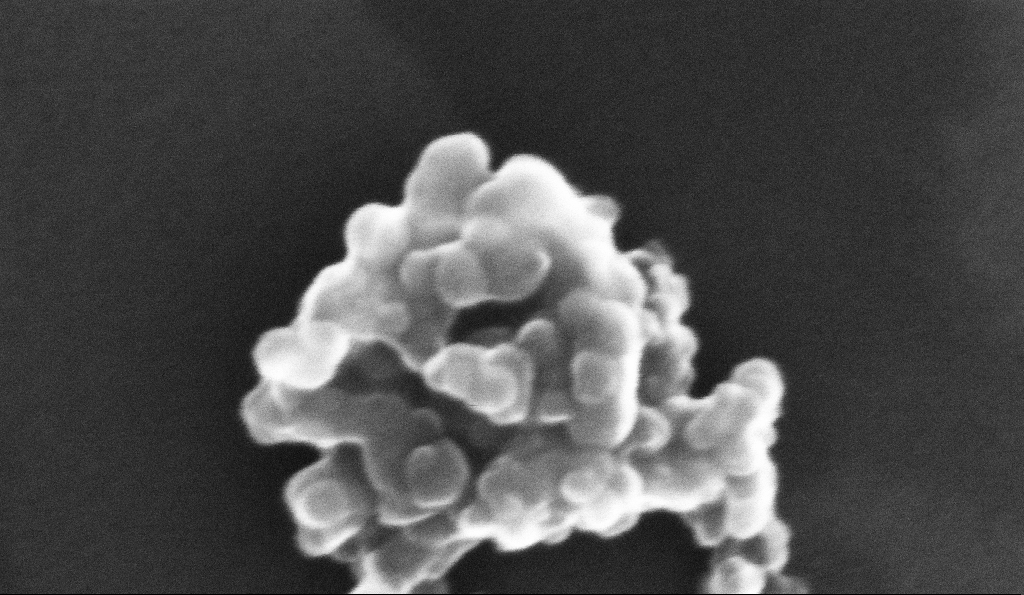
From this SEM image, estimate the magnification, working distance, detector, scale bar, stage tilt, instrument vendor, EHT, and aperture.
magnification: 500.87 K X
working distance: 5.3 mm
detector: InLens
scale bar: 100 nm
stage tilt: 0°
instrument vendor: Zeiss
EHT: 10 kV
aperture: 30 µm